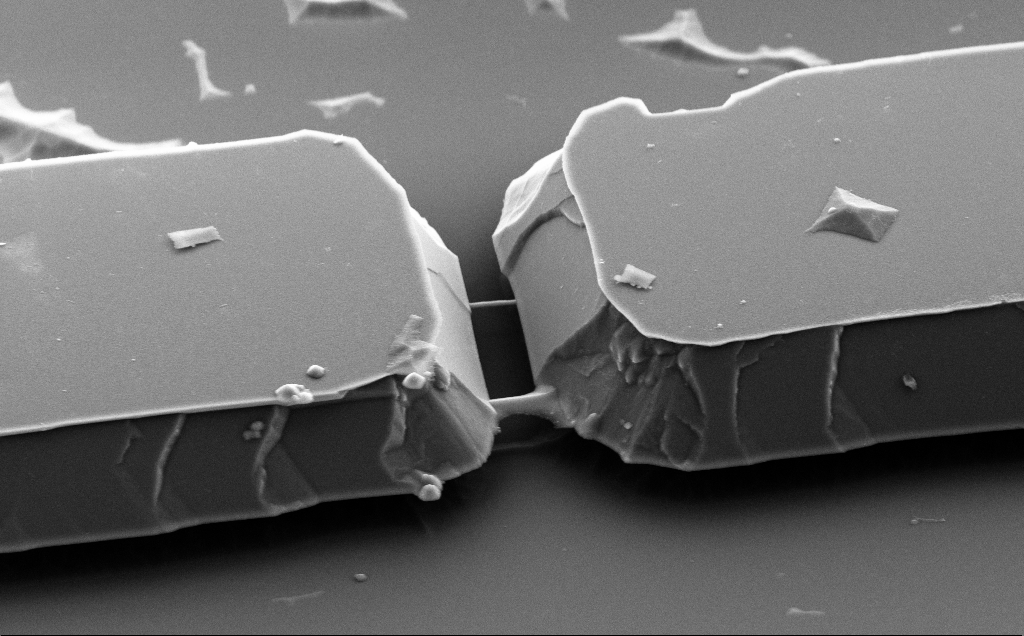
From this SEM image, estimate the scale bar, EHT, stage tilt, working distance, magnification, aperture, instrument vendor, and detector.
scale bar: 2000 nm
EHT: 5 kV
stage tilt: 50°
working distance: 10 mm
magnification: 9.88 K X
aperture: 30 µm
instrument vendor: Zeiss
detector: SE2